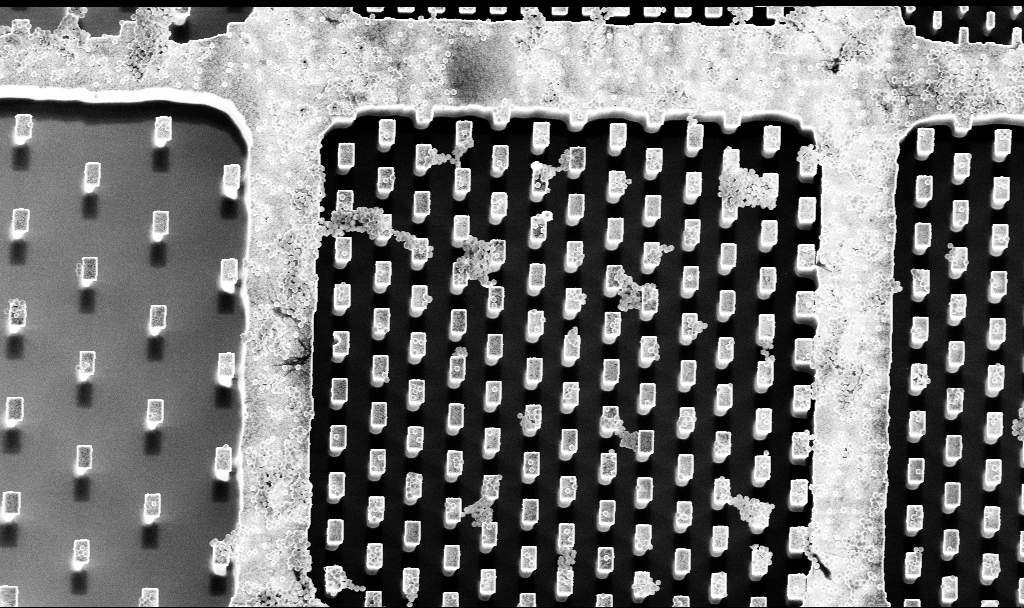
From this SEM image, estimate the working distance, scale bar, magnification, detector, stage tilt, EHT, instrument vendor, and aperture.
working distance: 3.3 mm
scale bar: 10000 nm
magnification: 3.59 K X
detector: InLens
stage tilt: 5°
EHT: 5 kV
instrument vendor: Zeiss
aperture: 30 µm